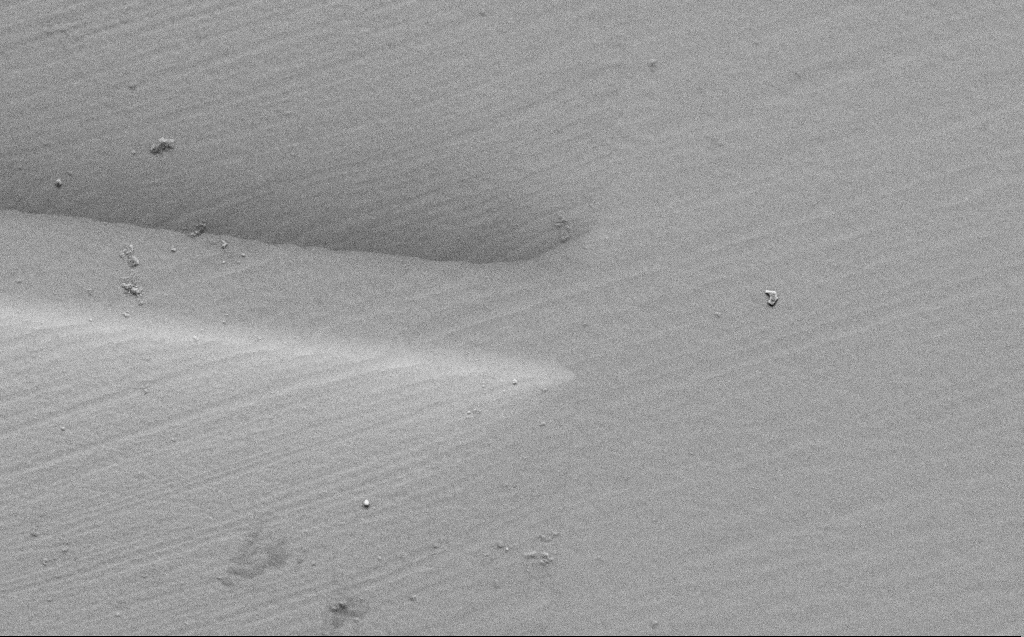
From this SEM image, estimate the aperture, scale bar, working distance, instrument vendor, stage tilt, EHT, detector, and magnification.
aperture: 30 µm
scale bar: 10000 nm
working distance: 8 mm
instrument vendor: Zeiss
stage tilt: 45°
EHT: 5 kV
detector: SE2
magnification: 2.92 K X